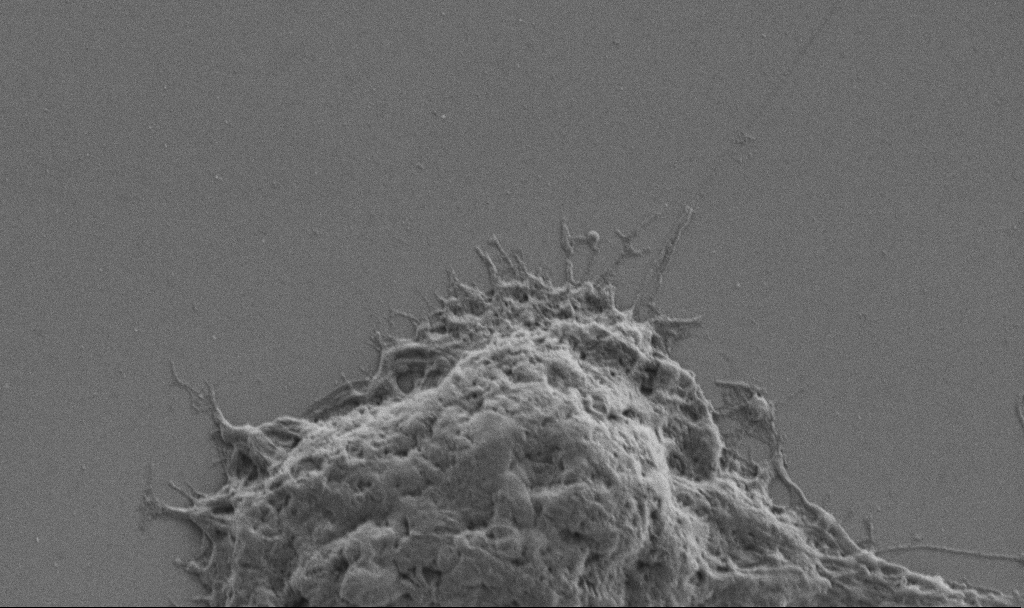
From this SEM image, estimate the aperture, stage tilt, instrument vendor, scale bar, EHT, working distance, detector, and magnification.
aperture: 30 µm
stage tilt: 0°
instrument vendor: Zeiss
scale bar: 2000 nm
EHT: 1 kV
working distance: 6.9 mm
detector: SE2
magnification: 10 K X